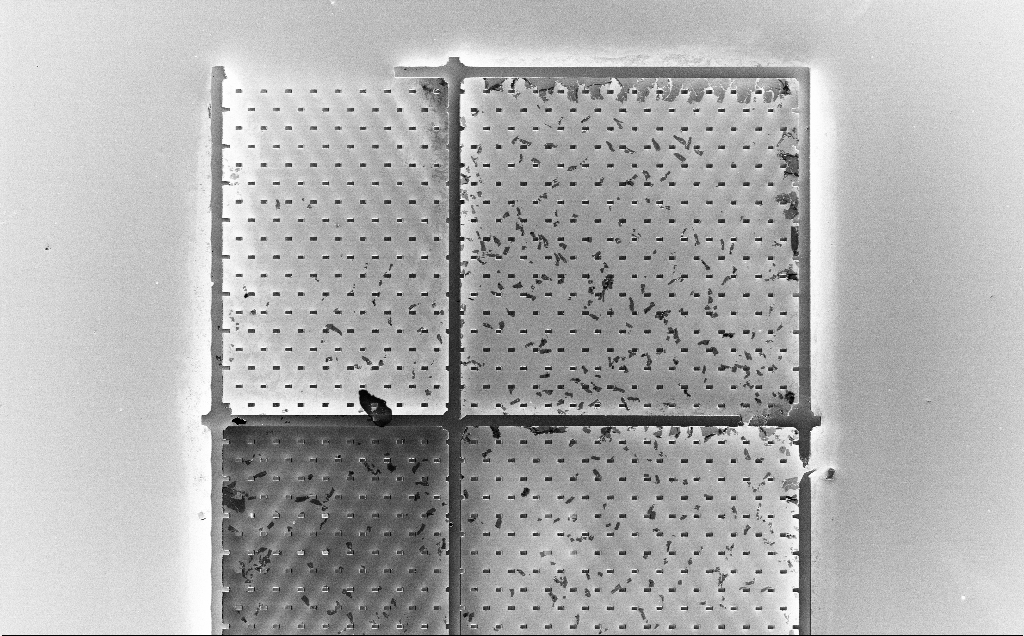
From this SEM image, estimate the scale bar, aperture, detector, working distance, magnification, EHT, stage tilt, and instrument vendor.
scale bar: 20000 nm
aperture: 30 µm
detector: InLens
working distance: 2.5 mm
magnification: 0.755 K X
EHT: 3 kV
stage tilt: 0°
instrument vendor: Zeiss